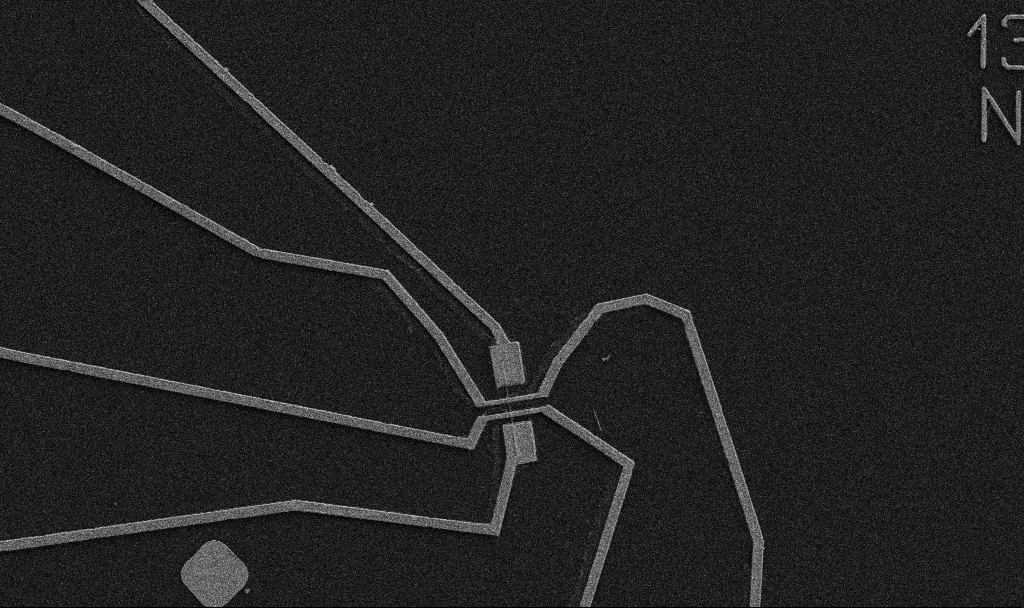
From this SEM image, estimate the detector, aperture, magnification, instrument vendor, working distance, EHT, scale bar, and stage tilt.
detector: SE2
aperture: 30 µm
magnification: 5 K X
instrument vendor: Zeiss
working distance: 10.7 mm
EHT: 5 kV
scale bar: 10000 nm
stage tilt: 0°